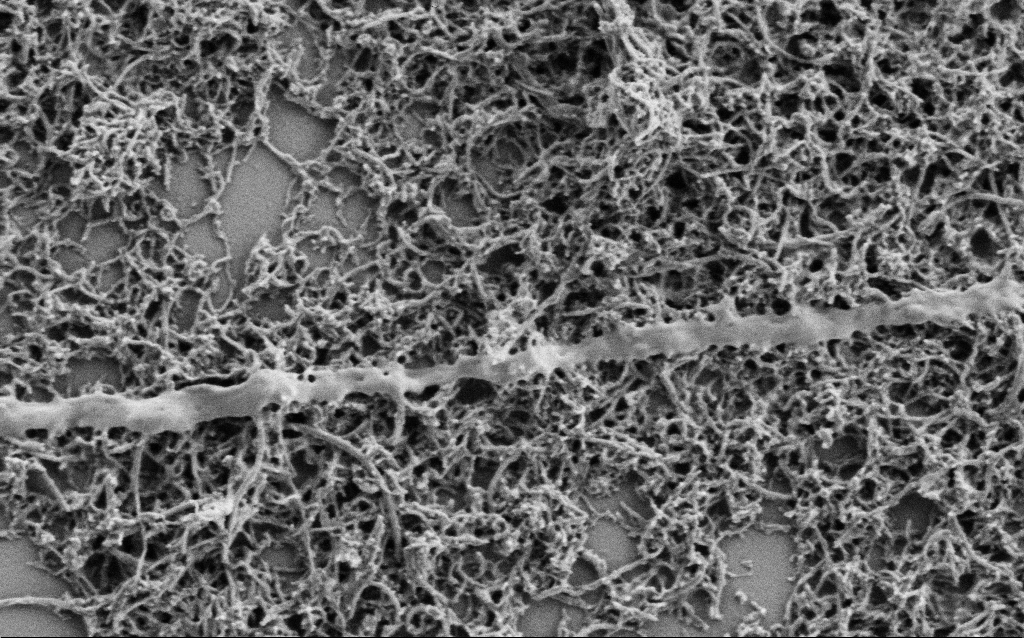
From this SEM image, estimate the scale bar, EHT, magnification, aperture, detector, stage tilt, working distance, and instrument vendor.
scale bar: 1000 nm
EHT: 1 kV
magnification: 50 K X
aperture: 30 µm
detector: SE2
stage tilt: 0°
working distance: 7 mm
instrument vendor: Zeiss